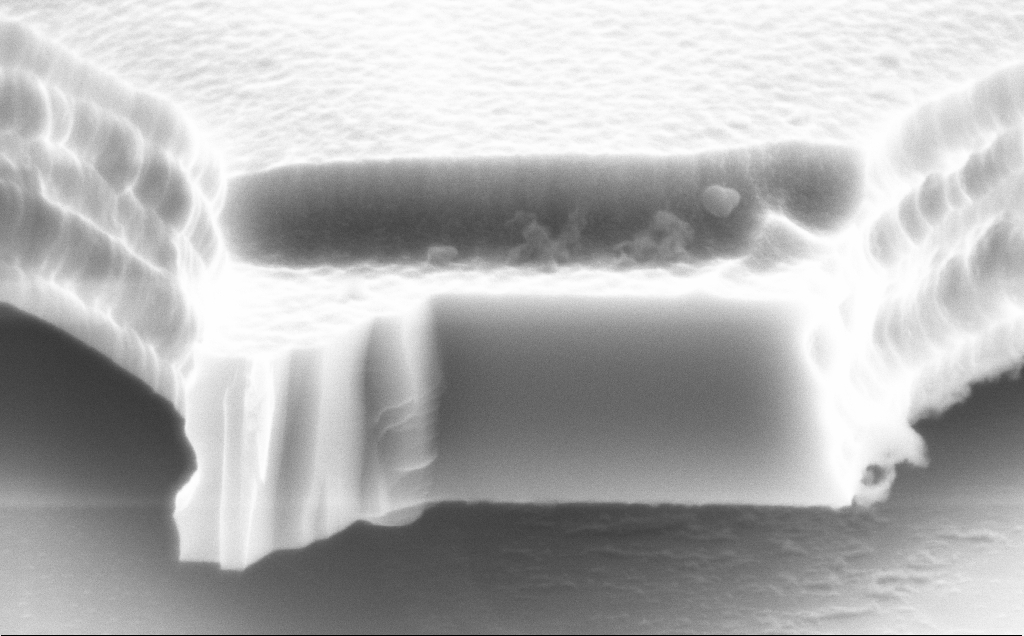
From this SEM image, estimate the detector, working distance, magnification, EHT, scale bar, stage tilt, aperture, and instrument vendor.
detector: SE2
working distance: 12 mm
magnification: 50.22 K X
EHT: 8 kV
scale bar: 1000 nm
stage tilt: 70°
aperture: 30 µm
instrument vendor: Zeiss